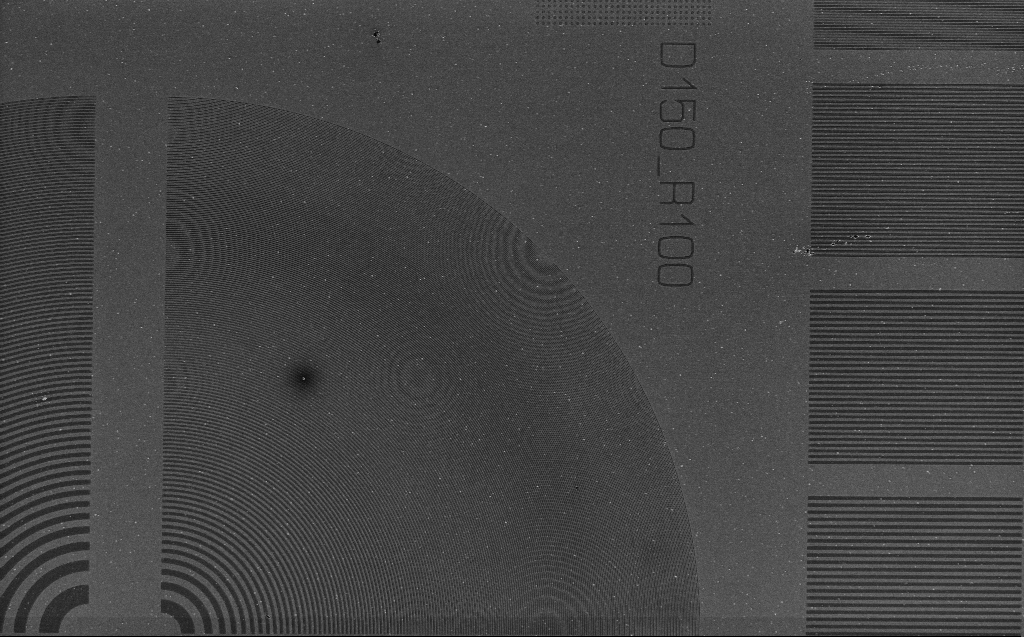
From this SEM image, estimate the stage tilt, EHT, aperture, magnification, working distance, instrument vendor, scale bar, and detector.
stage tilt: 0°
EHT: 1.2 kV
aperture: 30 µm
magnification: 2.64 K X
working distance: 5 mm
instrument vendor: Zeiss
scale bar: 10000 nm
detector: InLens